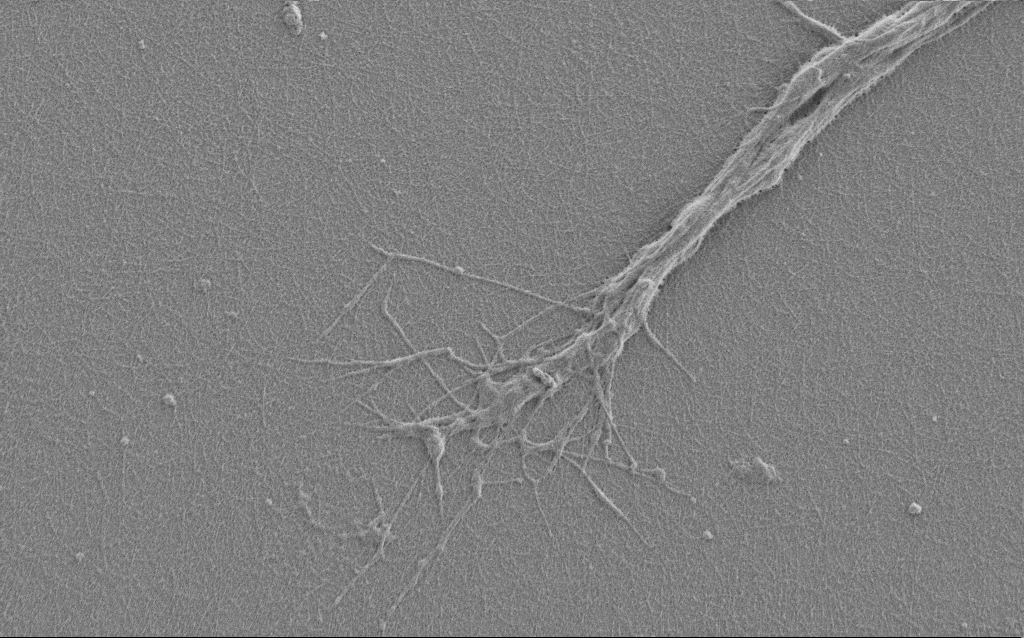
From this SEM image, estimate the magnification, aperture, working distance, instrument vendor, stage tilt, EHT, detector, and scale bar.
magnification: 7.5 K X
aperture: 30 µm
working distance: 6 mm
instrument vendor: Zeiss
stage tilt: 0°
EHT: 1 kV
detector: SE2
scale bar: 2000 nm